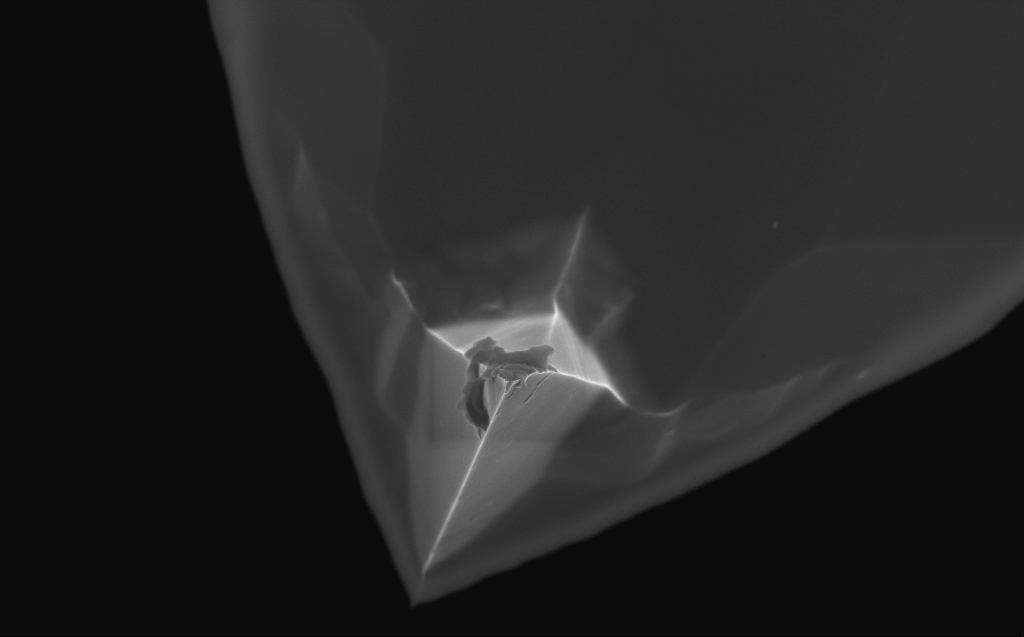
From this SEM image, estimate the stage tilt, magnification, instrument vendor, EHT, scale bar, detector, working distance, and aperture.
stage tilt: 0°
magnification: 9.38 K X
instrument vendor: Zeiss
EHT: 10 kV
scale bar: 2000 nm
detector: InLens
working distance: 4 mm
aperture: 30 µm